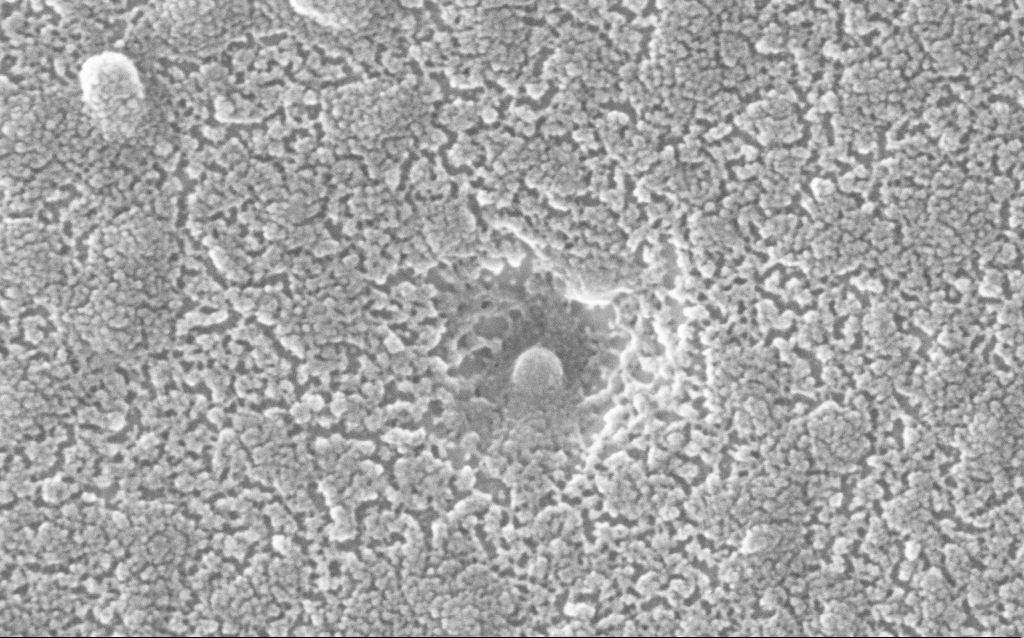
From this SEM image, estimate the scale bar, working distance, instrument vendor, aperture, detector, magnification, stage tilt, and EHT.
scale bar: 100 nm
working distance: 1.5 mm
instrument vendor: Zeiss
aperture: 30 µm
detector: InLens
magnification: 500 K X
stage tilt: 0°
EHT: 20 kV